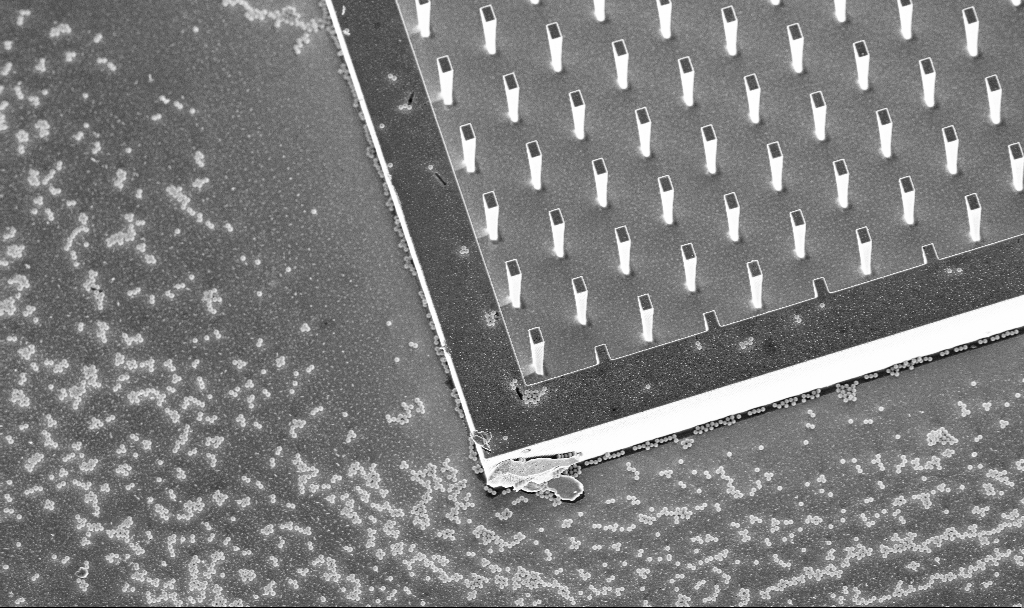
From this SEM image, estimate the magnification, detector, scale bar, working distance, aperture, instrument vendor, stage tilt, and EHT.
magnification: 2.28 K X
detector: InLens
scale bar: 10000 nm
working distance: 5.2 mm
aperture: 30 µm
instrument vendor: Zeiss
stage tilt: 20°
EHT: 5 kV